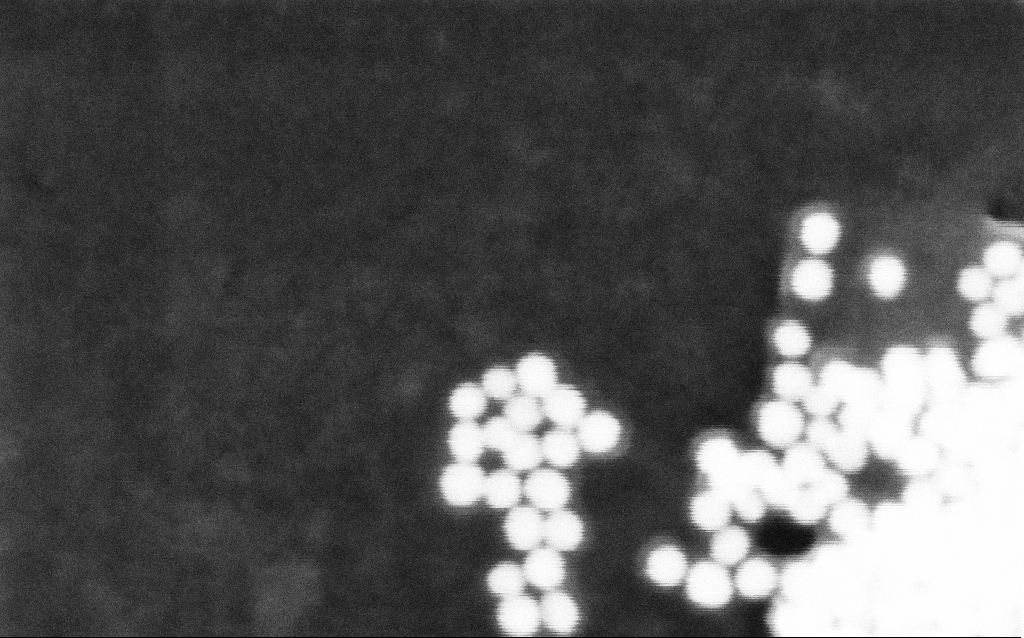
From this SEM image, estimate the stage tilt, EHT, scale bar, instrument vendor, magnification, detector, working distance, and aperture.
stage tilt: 0°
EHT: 30 kV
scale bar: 20 nm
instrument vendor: Zeiss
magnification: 725.74 K X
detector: SE2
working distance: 12.9 mm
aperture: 30 µm